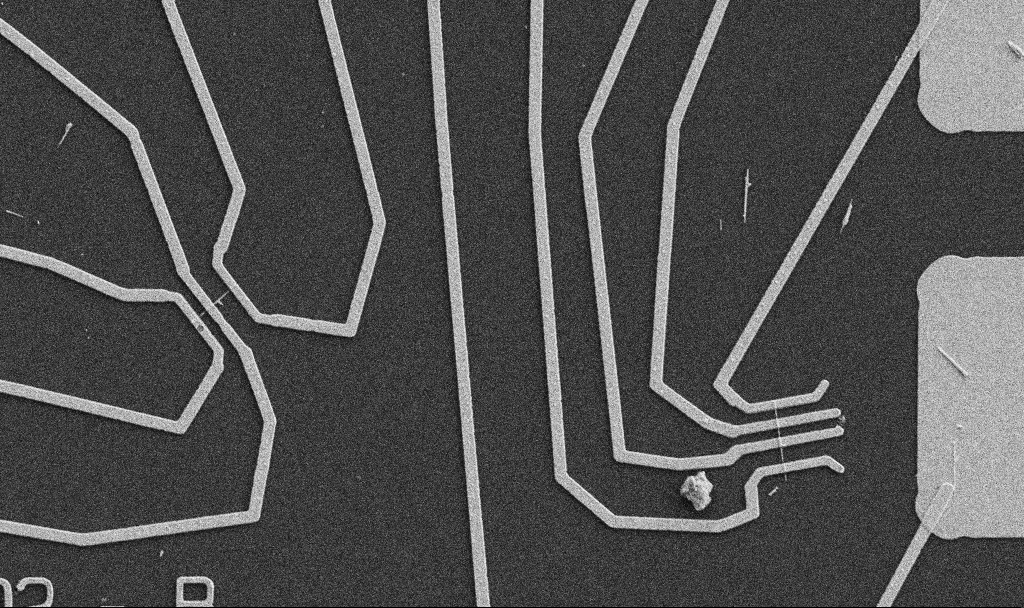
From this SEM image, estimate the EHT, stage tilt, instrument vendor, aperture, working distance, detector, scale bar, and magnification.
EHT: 5 kV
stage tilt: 0°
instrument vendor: Zeiss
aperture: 30 µm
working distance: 10.7 mm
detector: SE2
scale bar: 10000 nm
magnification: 5 K X